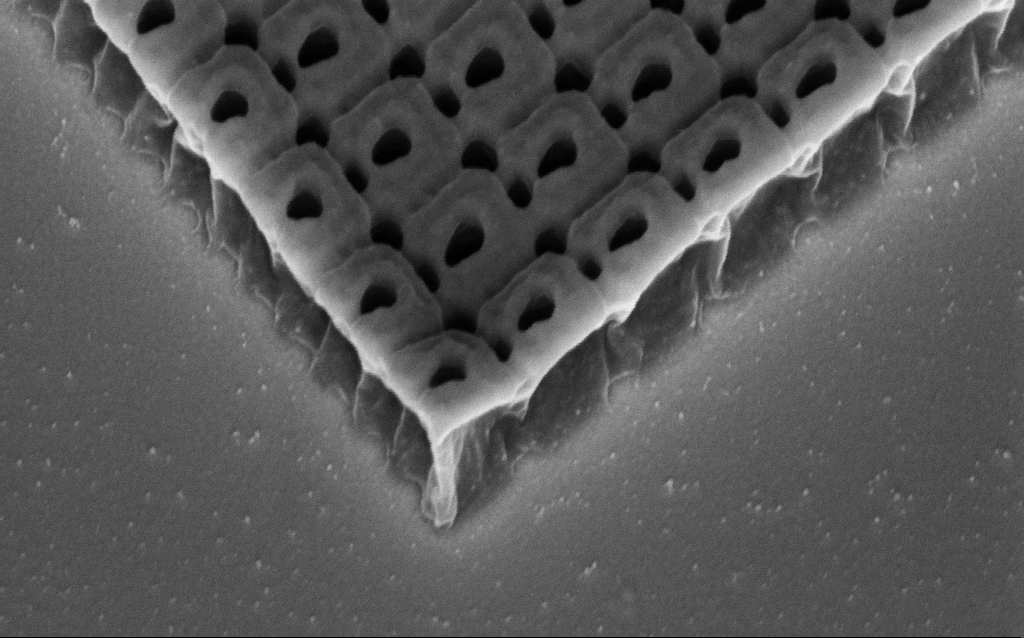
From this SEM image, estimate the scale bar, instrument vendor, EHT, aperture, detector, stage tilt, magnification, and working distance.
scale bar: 200 nm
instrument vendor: Zeiss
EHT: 3 kV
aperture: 30 µm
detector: InLens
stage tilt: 45°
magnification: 95.69 K X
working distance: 6.9 mm